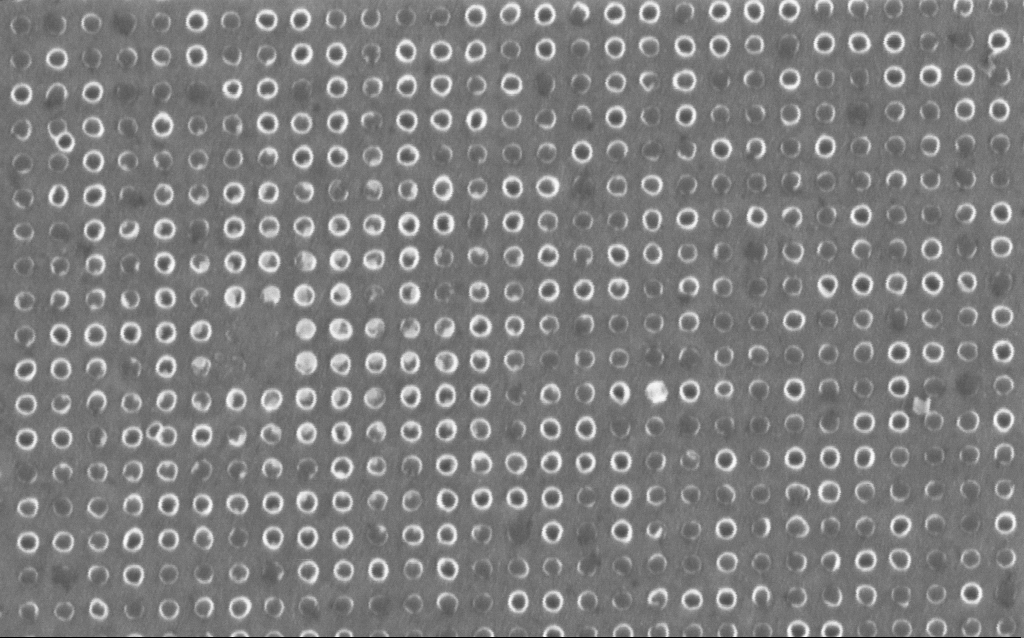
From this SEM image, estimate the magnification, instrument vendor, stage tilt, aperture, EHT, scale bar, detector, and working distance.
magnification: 90.76 K X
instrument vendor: Zeiss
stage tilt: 0°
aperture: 30 µm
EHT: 1.5 kV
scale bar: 200 nm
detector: InLens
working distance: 5.9 mm